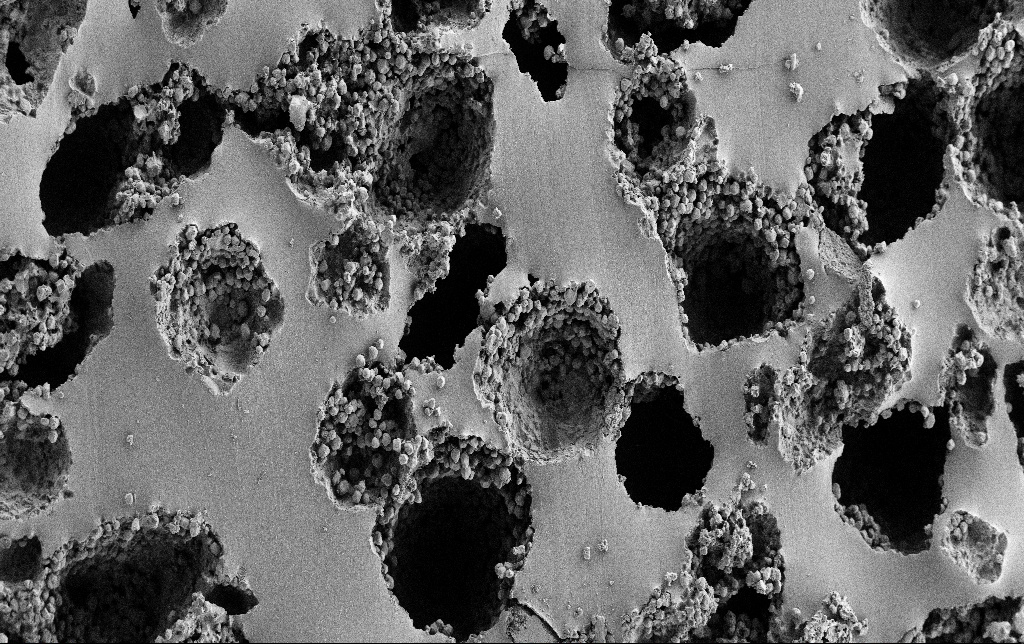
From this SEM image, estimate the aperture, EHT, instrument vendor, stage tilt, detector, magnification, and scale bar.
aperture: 30 µm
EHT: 3 kV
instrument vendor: Zeiss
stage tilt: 0°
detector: SE2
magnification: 0.25 K X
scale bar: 100000 nm